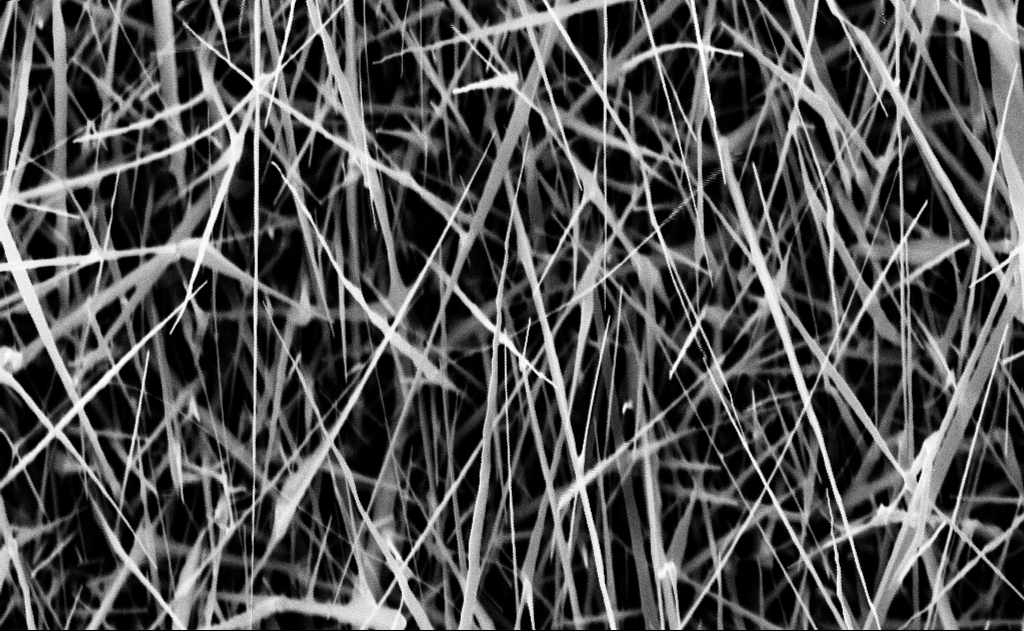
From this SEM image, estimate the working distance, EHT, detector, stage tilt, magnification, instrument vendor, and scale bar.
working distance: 17 mm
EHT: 10 kV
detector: InLens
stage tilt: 0°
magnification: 30 K X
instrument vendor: Zeiss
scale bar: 2000 nm